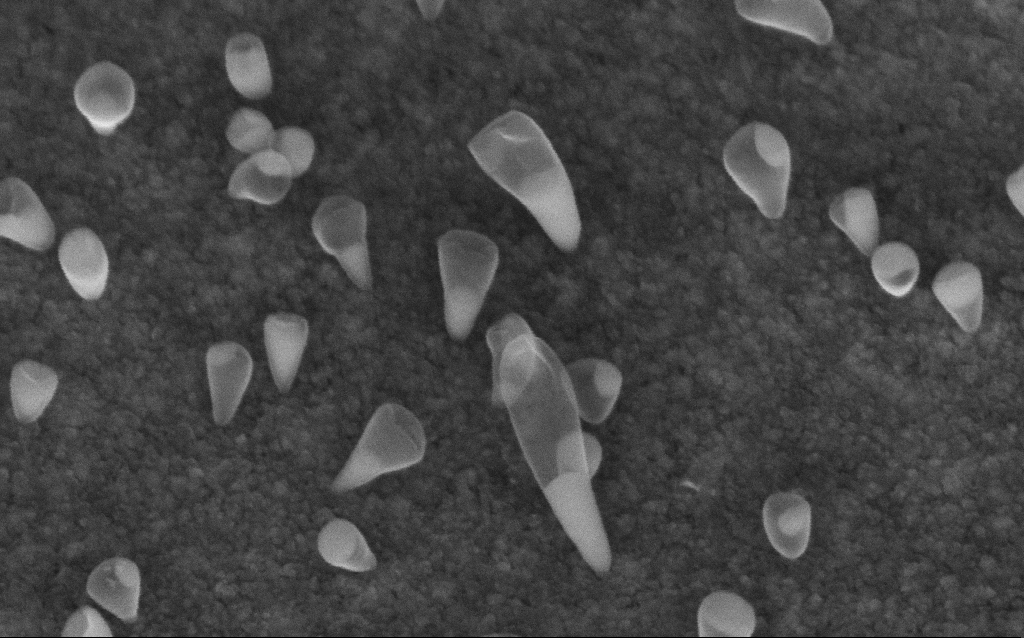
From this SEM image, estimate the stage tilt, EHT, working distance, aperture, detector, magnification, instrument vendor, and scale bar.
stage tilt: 45°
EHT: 5 kV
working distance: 6.6 mm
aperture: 30 µm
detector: InLens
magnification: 200 K X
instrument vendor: Zeiss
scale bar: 100 nm